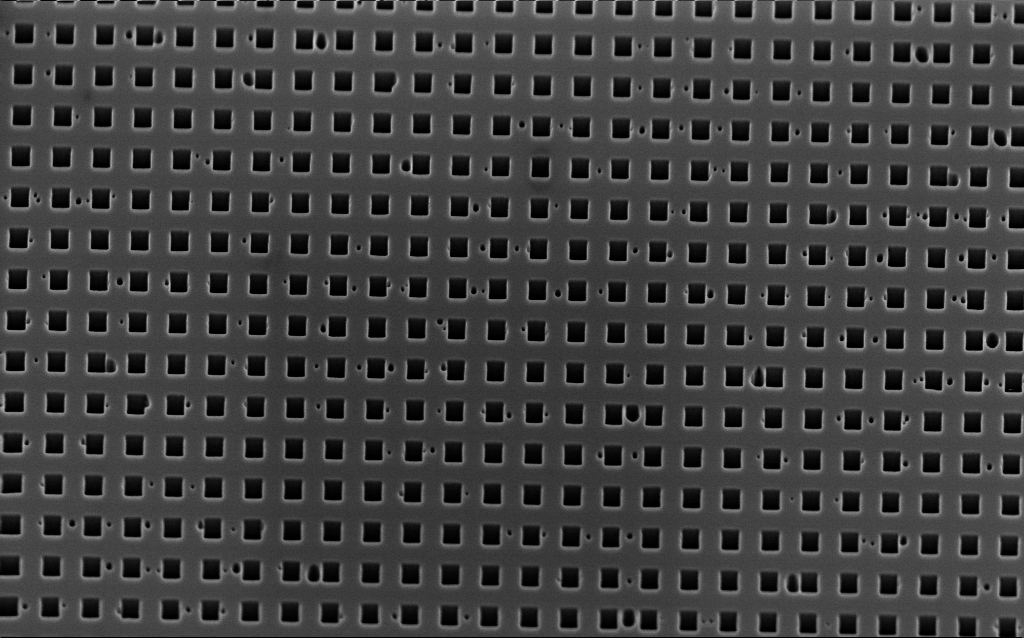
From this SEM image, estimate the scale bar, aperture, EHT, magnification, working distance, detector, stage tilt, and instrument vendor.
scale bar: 1000 nm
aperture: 30 µm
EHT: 2 kV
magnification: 29.47 K X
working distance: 3.6 mm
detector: InLens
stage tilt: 45°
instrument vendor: Zeiss